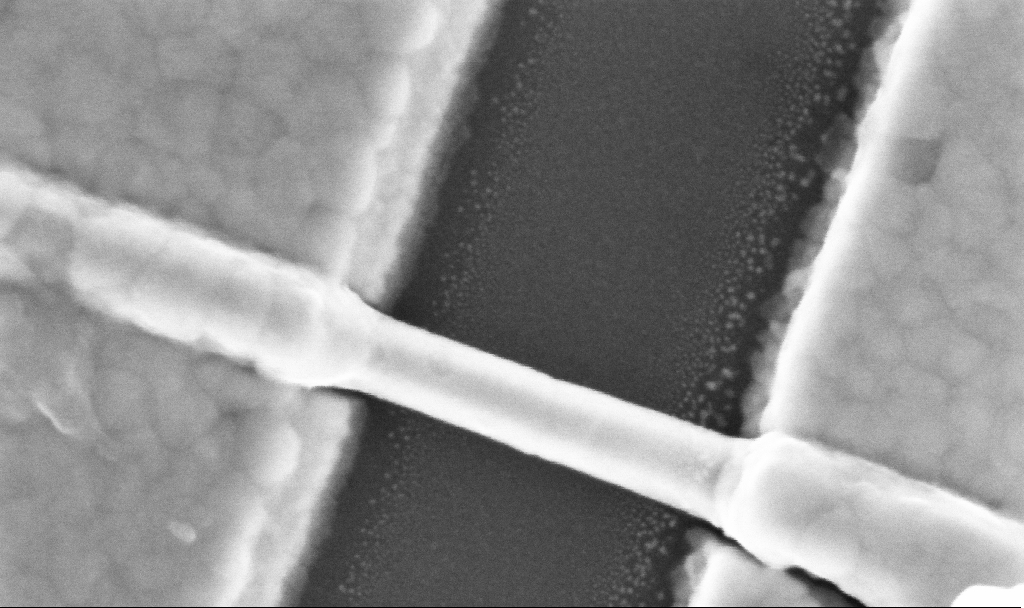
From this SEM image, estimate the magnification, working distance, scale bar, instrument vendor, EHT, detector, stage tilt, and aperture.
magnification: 300 K X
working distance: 6.7 mm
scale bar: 200 nm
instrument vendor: Zeiss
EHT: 5 kV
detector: InLens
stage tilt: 0°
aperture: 30 µm